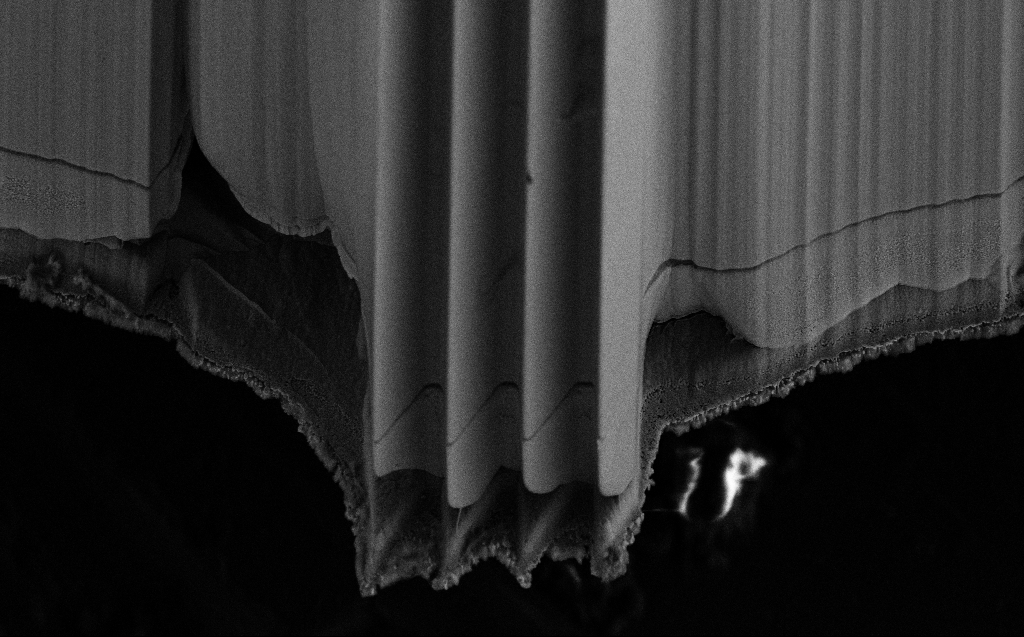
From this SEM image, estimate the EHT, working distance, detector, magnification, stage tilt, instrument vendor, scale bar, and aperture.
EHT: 10 kV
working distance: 3 mm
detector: SE2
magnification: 2.44 K X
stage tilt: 45°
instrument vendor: Zeiss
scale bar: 10000 nm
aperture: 30 µm